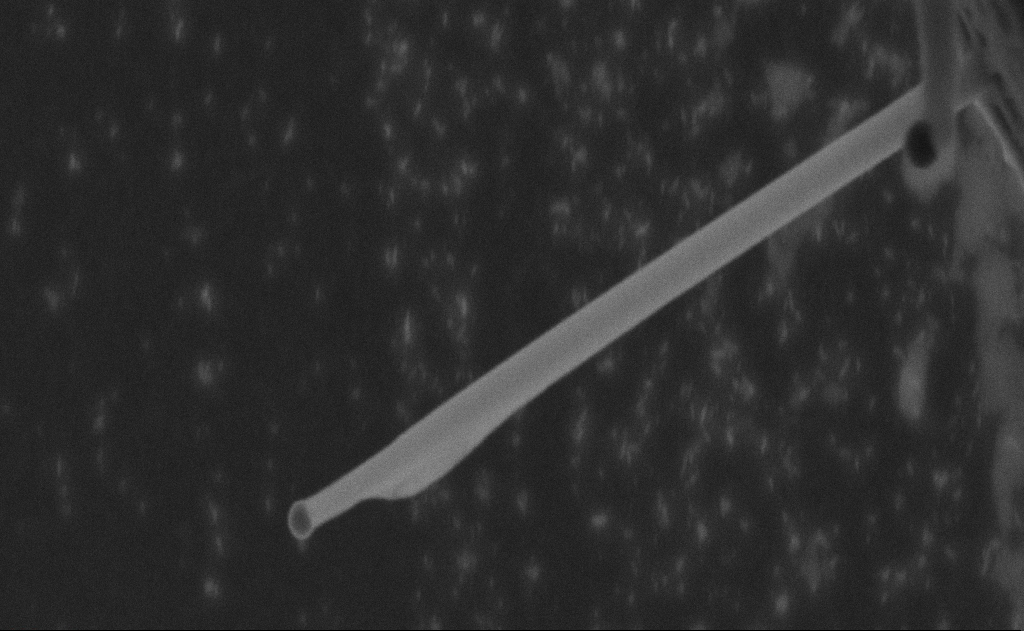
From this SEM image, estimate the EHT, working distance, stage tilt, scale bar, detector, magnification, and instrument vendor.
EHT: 20 kV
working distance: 9 mm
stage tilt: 0°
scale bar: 200 nm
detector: SE2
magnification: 185.57 K X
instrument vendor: Zeiss